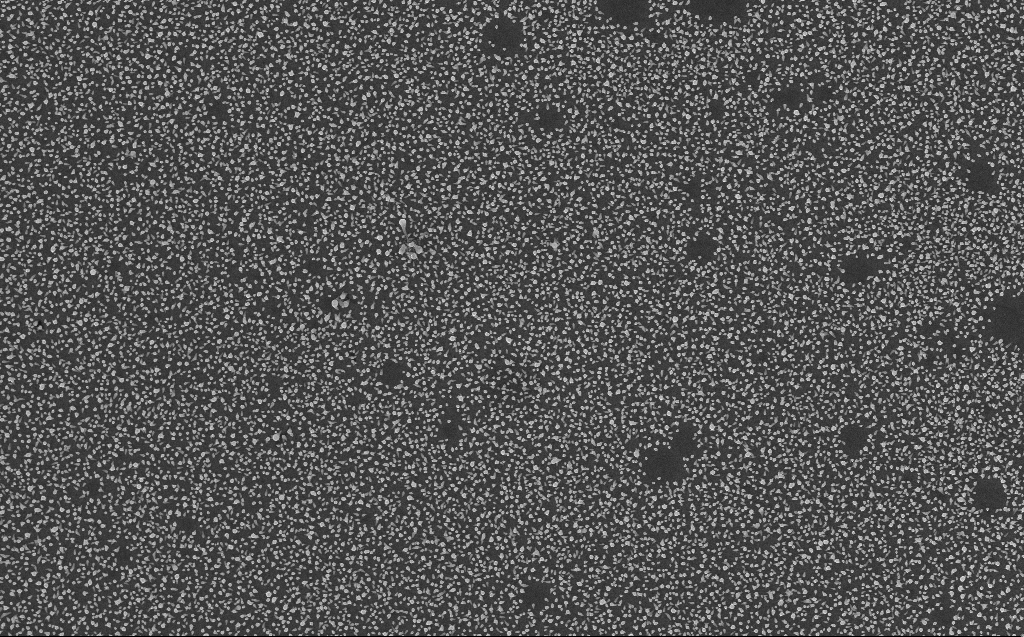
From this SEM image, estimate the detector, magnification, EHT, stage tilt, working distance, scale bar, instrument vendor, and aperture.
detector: InLens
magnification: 10 K X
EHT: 10 kV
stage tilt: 0°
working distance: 3 mm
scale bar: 2000 nm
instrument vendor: Zeiss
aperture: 30 µm